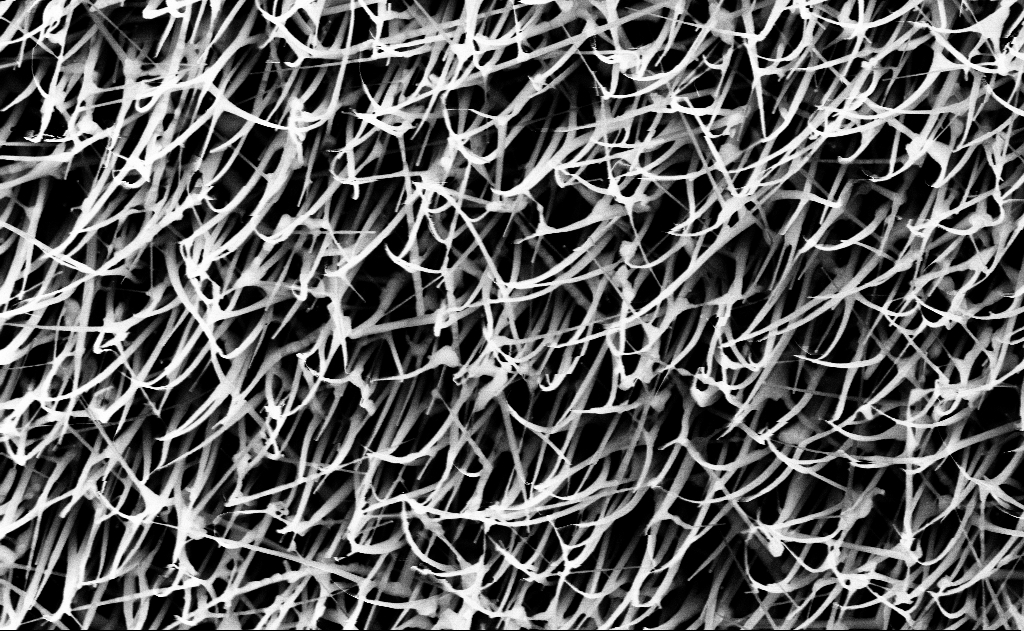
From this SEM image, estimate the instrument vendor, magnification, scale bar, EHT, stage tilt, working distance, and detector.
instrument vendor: Zeiss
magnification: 40 K X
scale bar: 1000 nm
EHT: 10 kV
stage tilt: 0°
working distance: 12 mm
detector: InLens